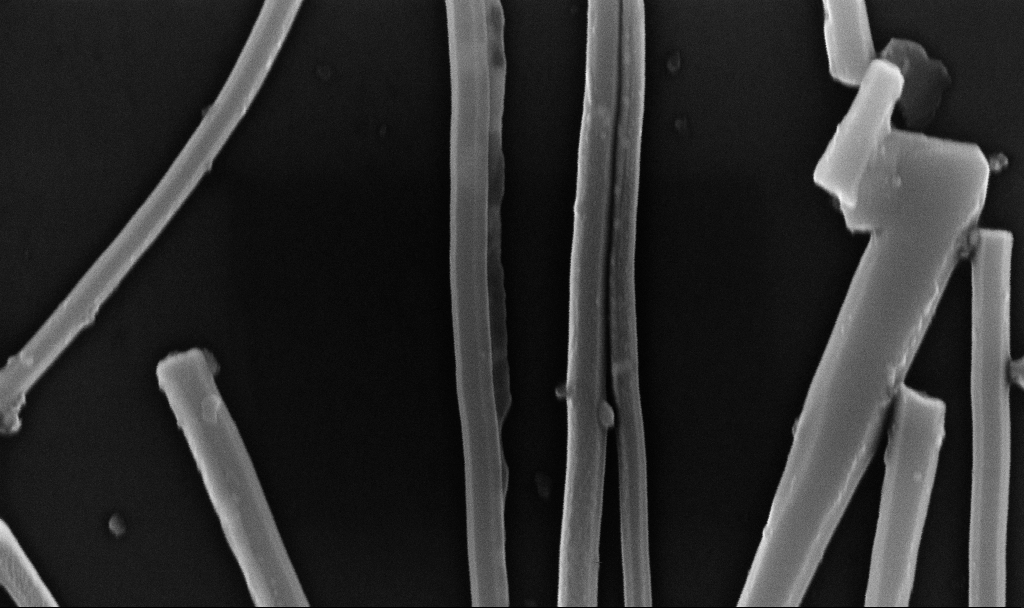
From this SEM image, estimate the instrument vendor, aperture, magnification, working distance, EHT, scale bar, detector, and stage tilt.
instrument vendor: Zeiss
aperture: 30 µm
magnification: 121.84 K X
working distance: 6.7 mm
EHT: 10 kV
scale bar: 200 nm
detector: InLens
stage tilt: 0°